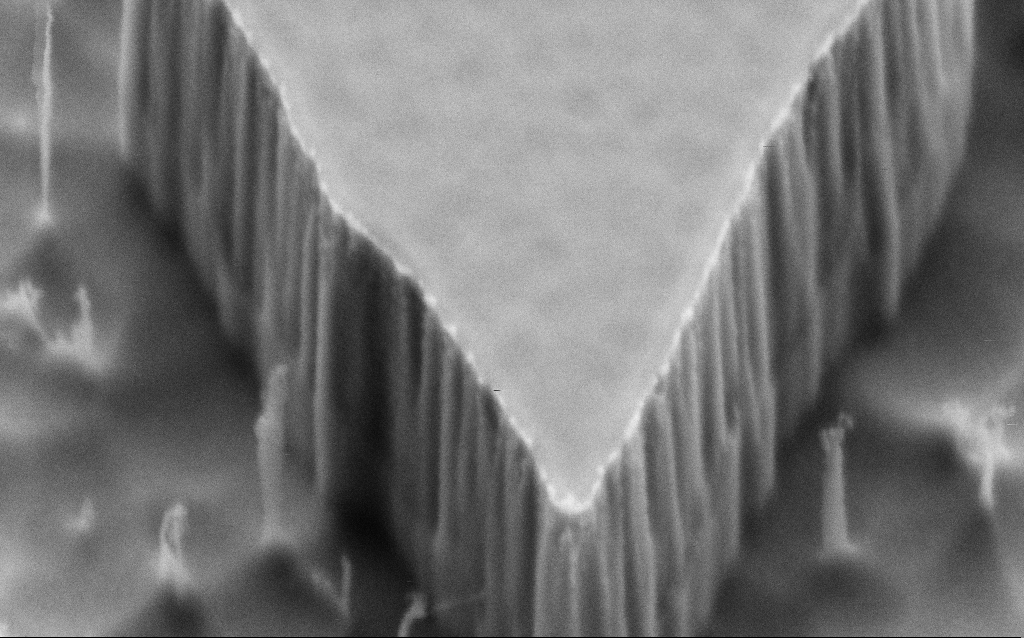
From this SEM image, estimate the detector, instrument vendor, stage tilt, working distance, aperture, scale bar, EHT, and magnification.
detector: SE2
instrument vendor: Zeiss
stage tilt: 45°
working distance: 6 mm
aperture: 30 µm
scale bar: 100 nm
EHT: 2 kV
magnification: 184.94 K X